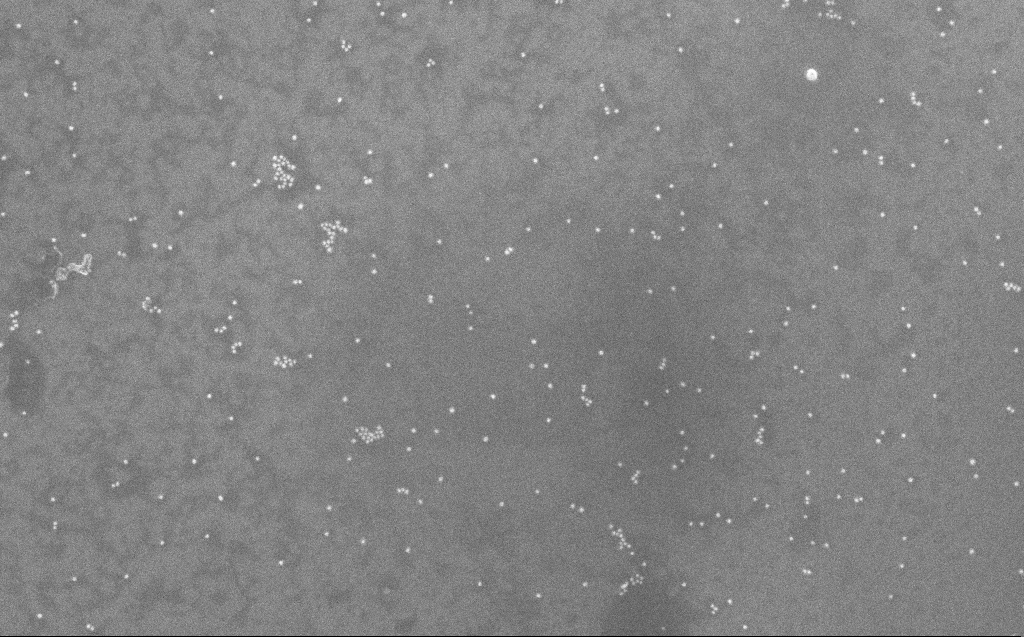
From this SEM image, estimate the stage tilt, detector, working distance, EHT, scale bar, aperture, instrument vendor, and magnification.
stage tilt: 0°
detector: InLens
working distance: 3 mm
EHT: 10 kV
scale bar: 200 nm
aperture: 30 µm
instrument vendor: Zeiss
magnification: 81.84 K X